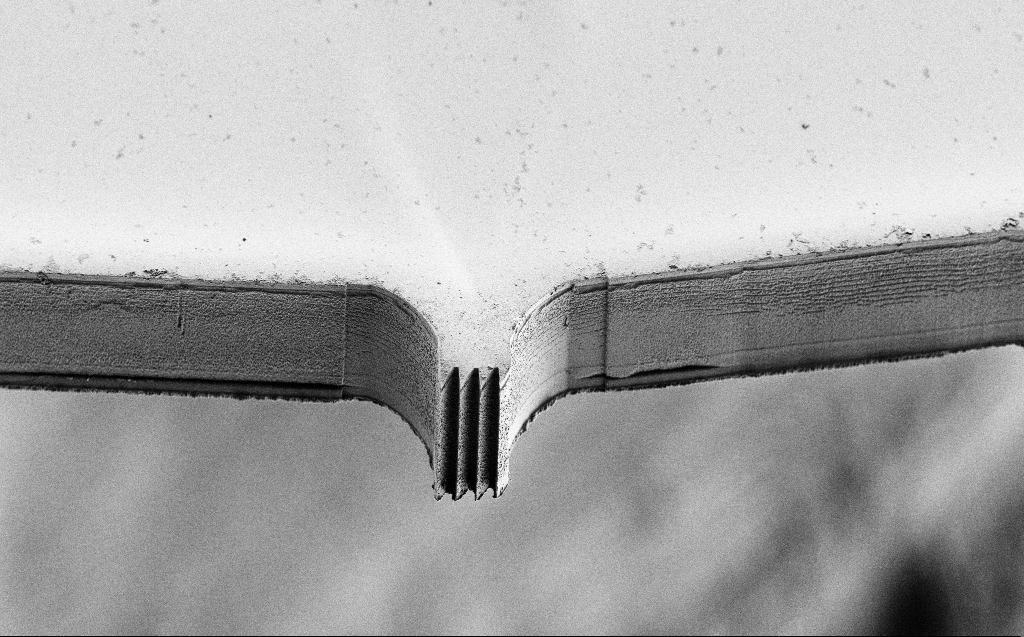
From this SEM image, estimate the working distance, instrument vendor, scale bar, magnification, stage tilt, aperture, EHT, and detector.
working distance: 6 mm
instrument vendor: Zeiss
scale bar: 100000 nm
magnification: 0.652 K X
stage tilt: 45°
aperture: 30 µm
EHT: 3 kV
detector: SE2